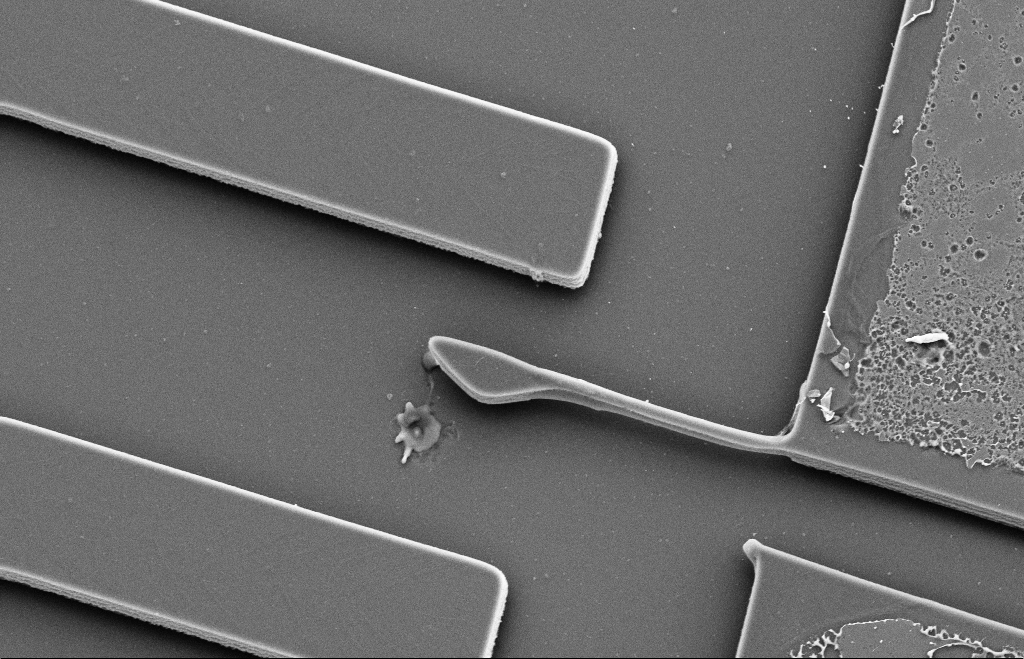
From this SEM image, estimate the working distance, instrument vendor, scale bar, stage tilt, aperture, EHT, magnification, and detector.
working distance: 11 mm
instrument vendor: Zeiss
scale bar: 10000 nm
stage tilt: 15.3°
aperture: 30 µm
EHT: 10 kV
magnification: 2.77 K X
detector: SE2